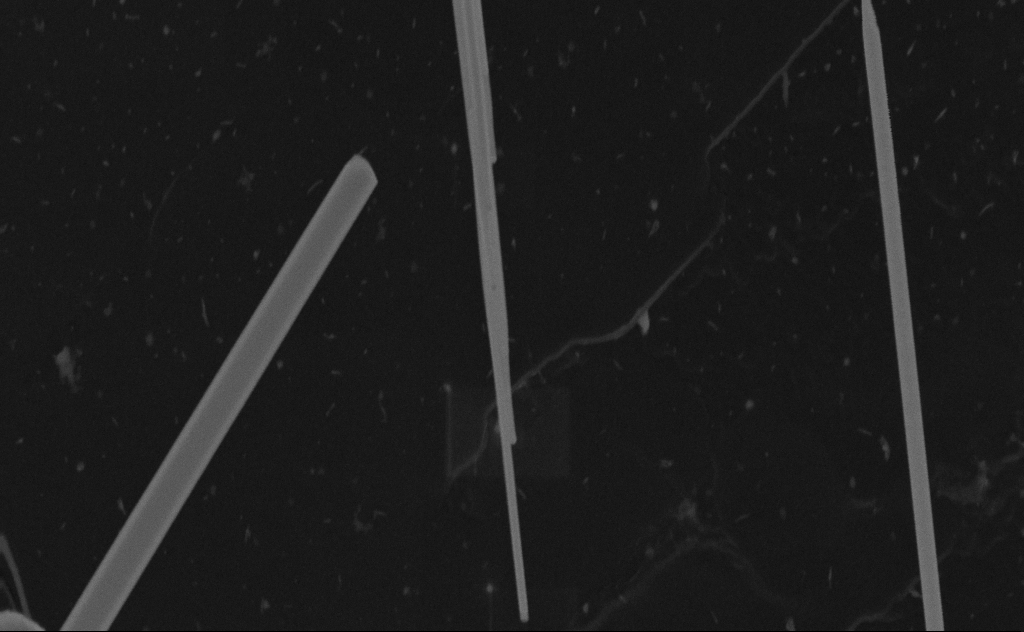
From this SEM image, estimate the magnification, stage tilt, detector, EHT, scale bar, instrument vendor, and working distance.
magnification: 104.22 K X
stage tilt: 0°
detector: SE2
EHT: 20 kV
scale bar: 200 nm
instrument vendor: Zeiss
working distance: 8 mm